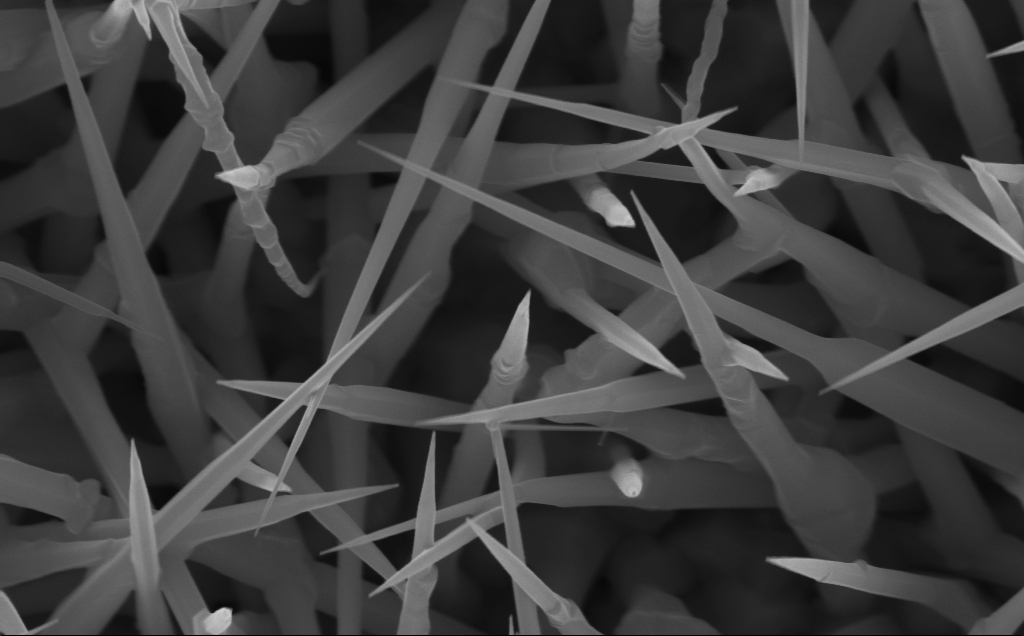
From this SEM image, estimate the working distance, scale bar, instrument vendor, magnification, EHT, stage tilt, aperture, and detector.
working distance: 4 mm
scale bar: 200 nm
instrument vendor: Zeiss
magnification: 80 K X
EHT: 10 kV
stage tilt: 0°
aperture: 30 µm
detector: InLens